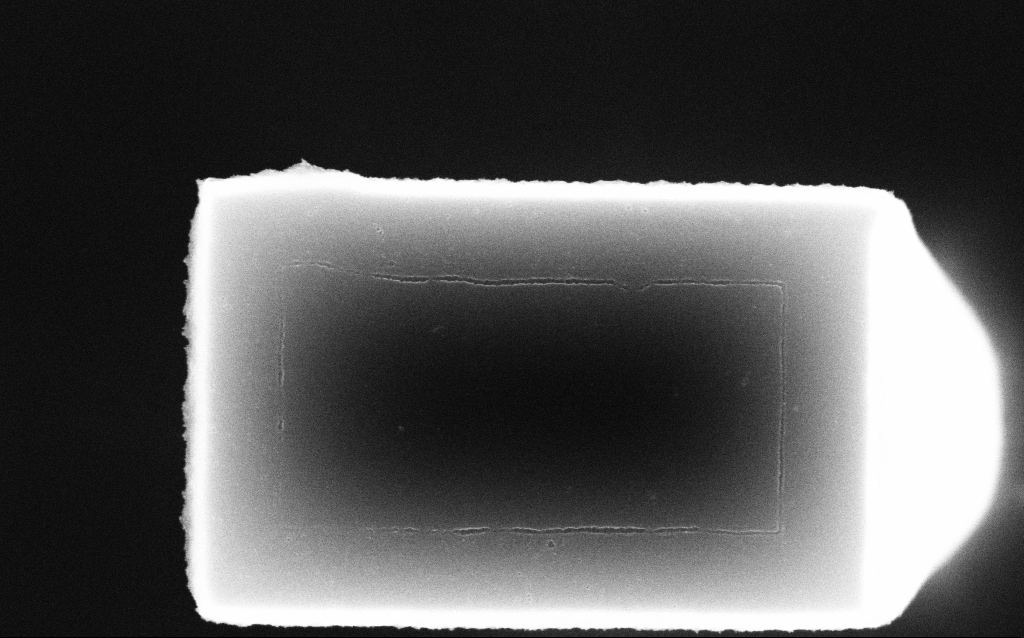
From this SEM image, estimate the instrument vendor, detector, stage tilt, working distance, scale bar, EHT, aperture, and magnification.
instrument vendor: Zeiss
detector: InLens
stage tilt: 0°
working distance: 8.3 mm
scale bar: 200 nm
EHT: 10 kV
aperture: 30 µm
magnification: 89.21 K X